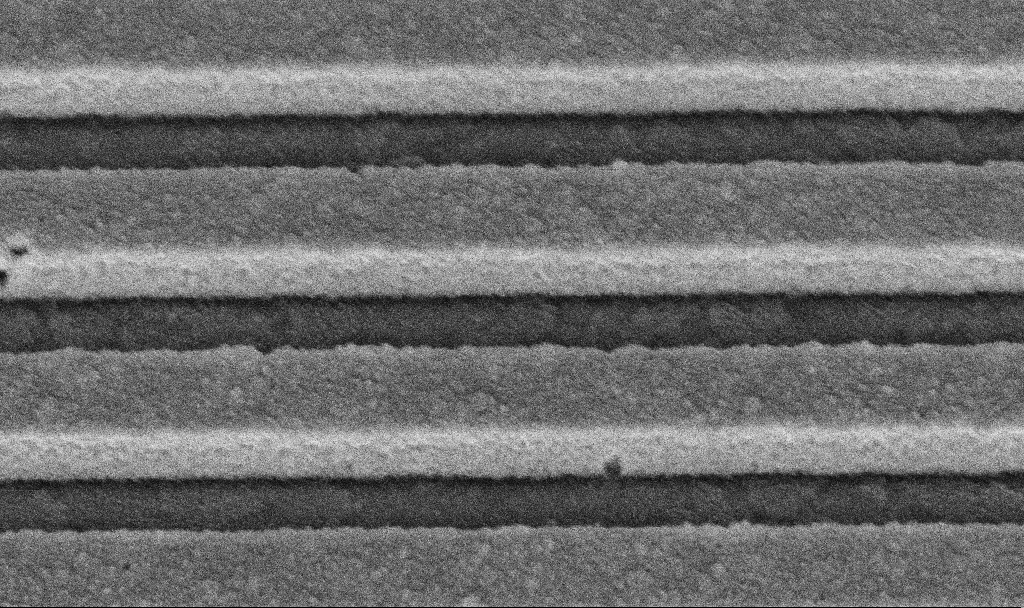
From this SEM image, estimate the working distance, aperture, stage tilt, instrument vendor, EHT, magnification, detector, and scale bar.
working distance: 8.7 mm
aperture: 30 µm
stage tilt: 45°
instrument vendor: Zeiss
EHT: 5 kV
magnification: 111.71 K X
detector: InLens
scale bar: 200 nm